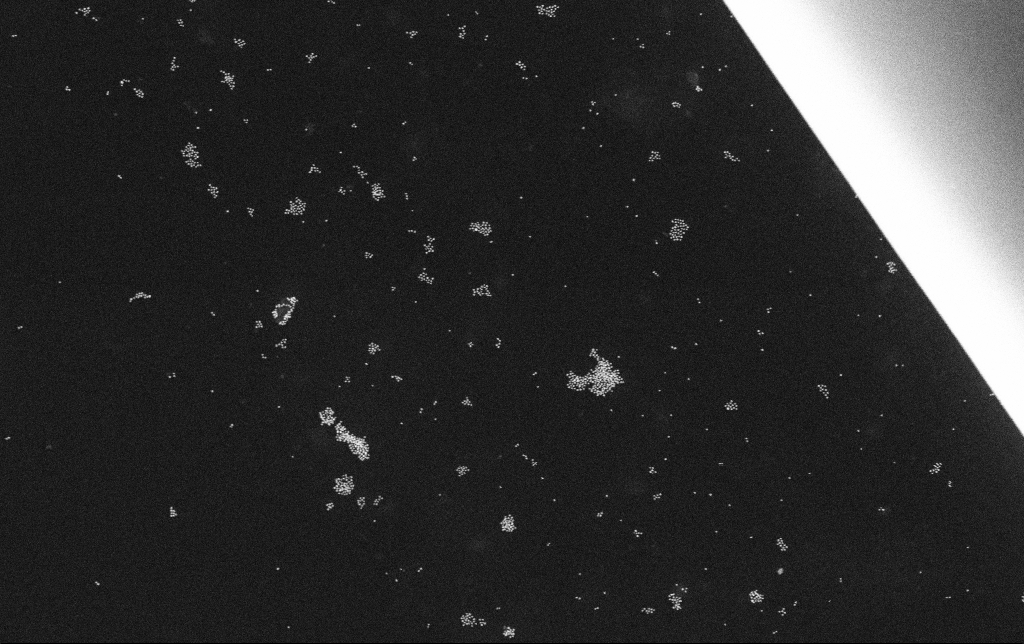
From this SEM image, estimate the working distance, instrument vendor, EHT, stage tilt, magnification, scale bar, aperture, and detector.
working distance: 10.8 mm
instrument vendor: Zeiss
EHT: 30 kV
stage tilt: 0°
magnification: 63.92 K X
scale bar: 1000 nm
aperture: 30 µm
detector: SE2